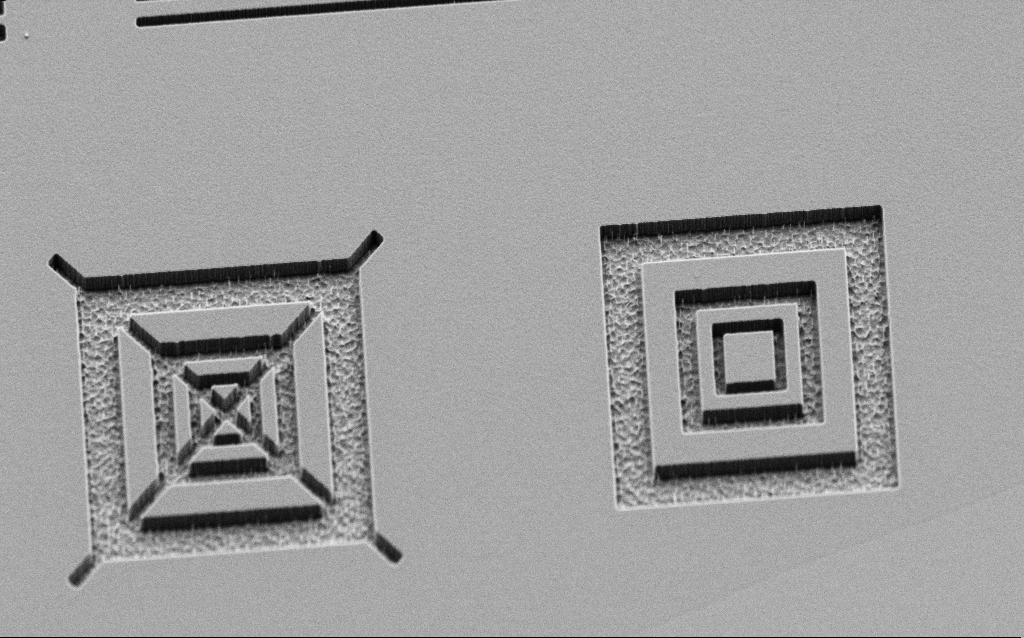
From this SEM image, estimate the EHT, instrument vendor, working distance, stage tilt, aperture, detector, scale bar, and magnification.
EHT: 2 kV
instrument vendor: Zeiss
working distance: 6 mm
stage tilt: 45°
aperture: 30 µm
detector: SE2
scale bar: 2000 nm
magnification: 9.49 K X